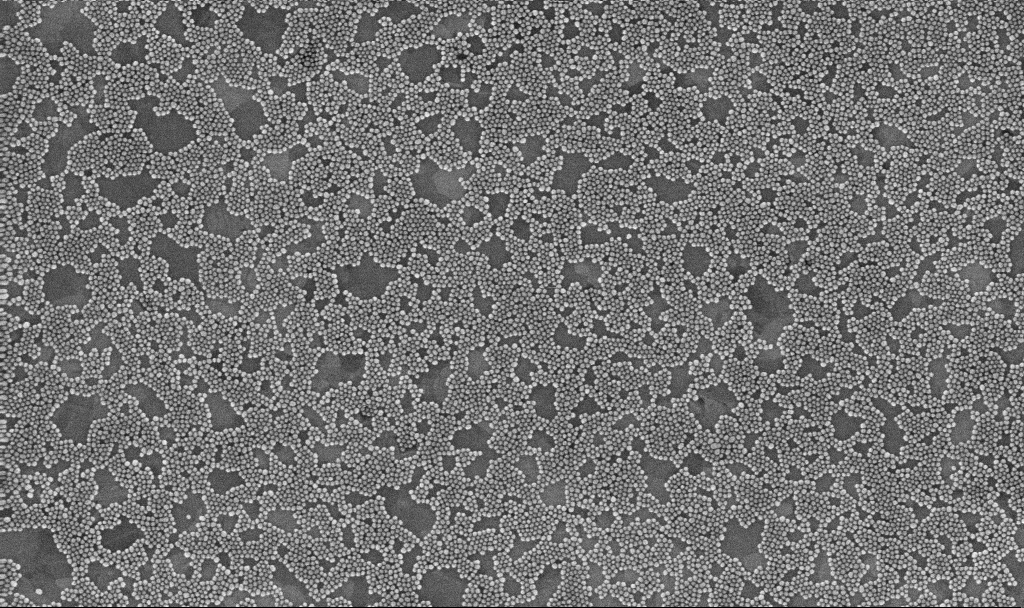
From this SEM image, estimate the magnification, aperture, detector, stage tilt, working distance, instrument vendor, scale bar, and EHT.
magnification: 100 K X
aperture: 30 µm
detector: InLens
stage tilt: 0°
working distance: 3.4 mm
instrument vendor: Zeiss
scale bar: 200 nm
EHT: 10 kV